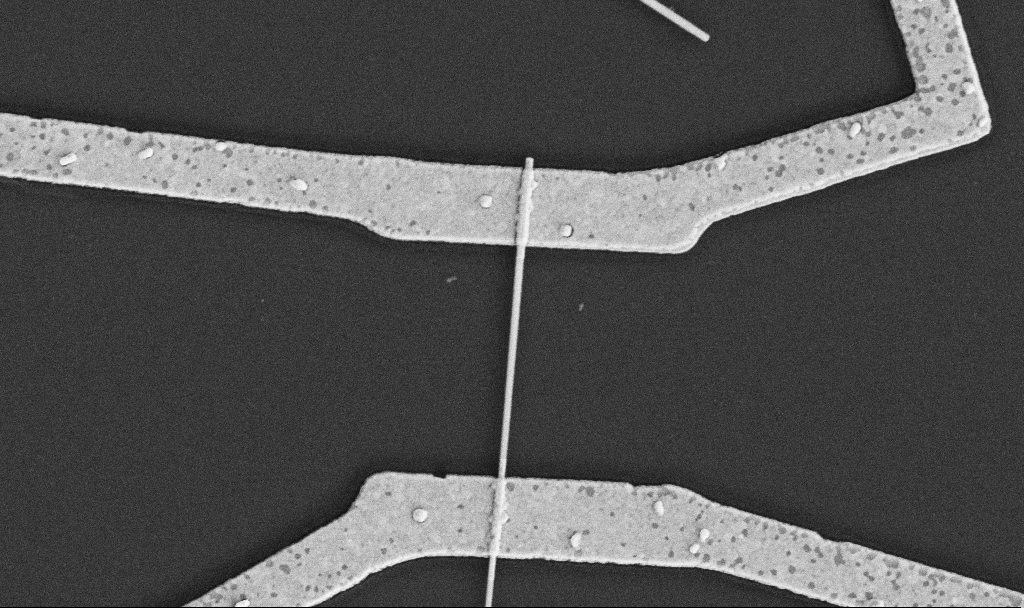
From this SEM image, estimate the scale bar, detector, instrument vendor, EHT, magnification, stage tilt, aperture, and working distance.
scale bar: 1000 nm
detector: SE2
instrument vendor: Zeiss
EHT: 5 kV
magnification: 30 K X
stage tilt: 0°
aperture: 30 µm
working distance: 10.6 mm